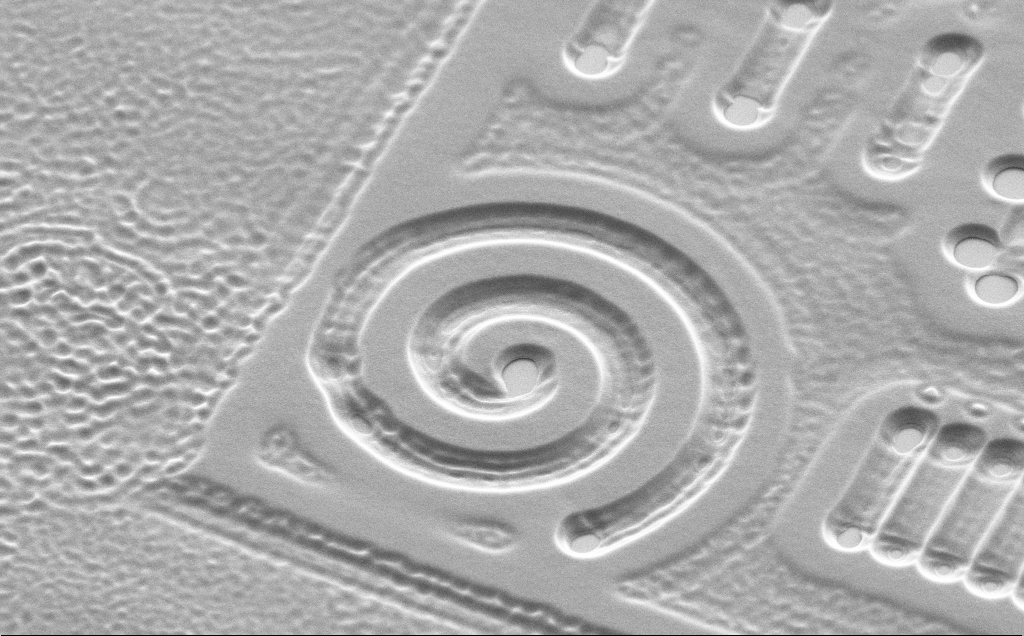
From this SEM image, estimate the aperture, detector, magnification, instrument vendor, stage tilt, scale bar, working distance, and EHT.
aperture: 30 µm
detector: SE2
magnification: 5.96 K X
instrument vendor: Zeiss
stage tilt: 45°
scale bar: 10000 nm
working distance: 10 mm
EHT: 5 kV